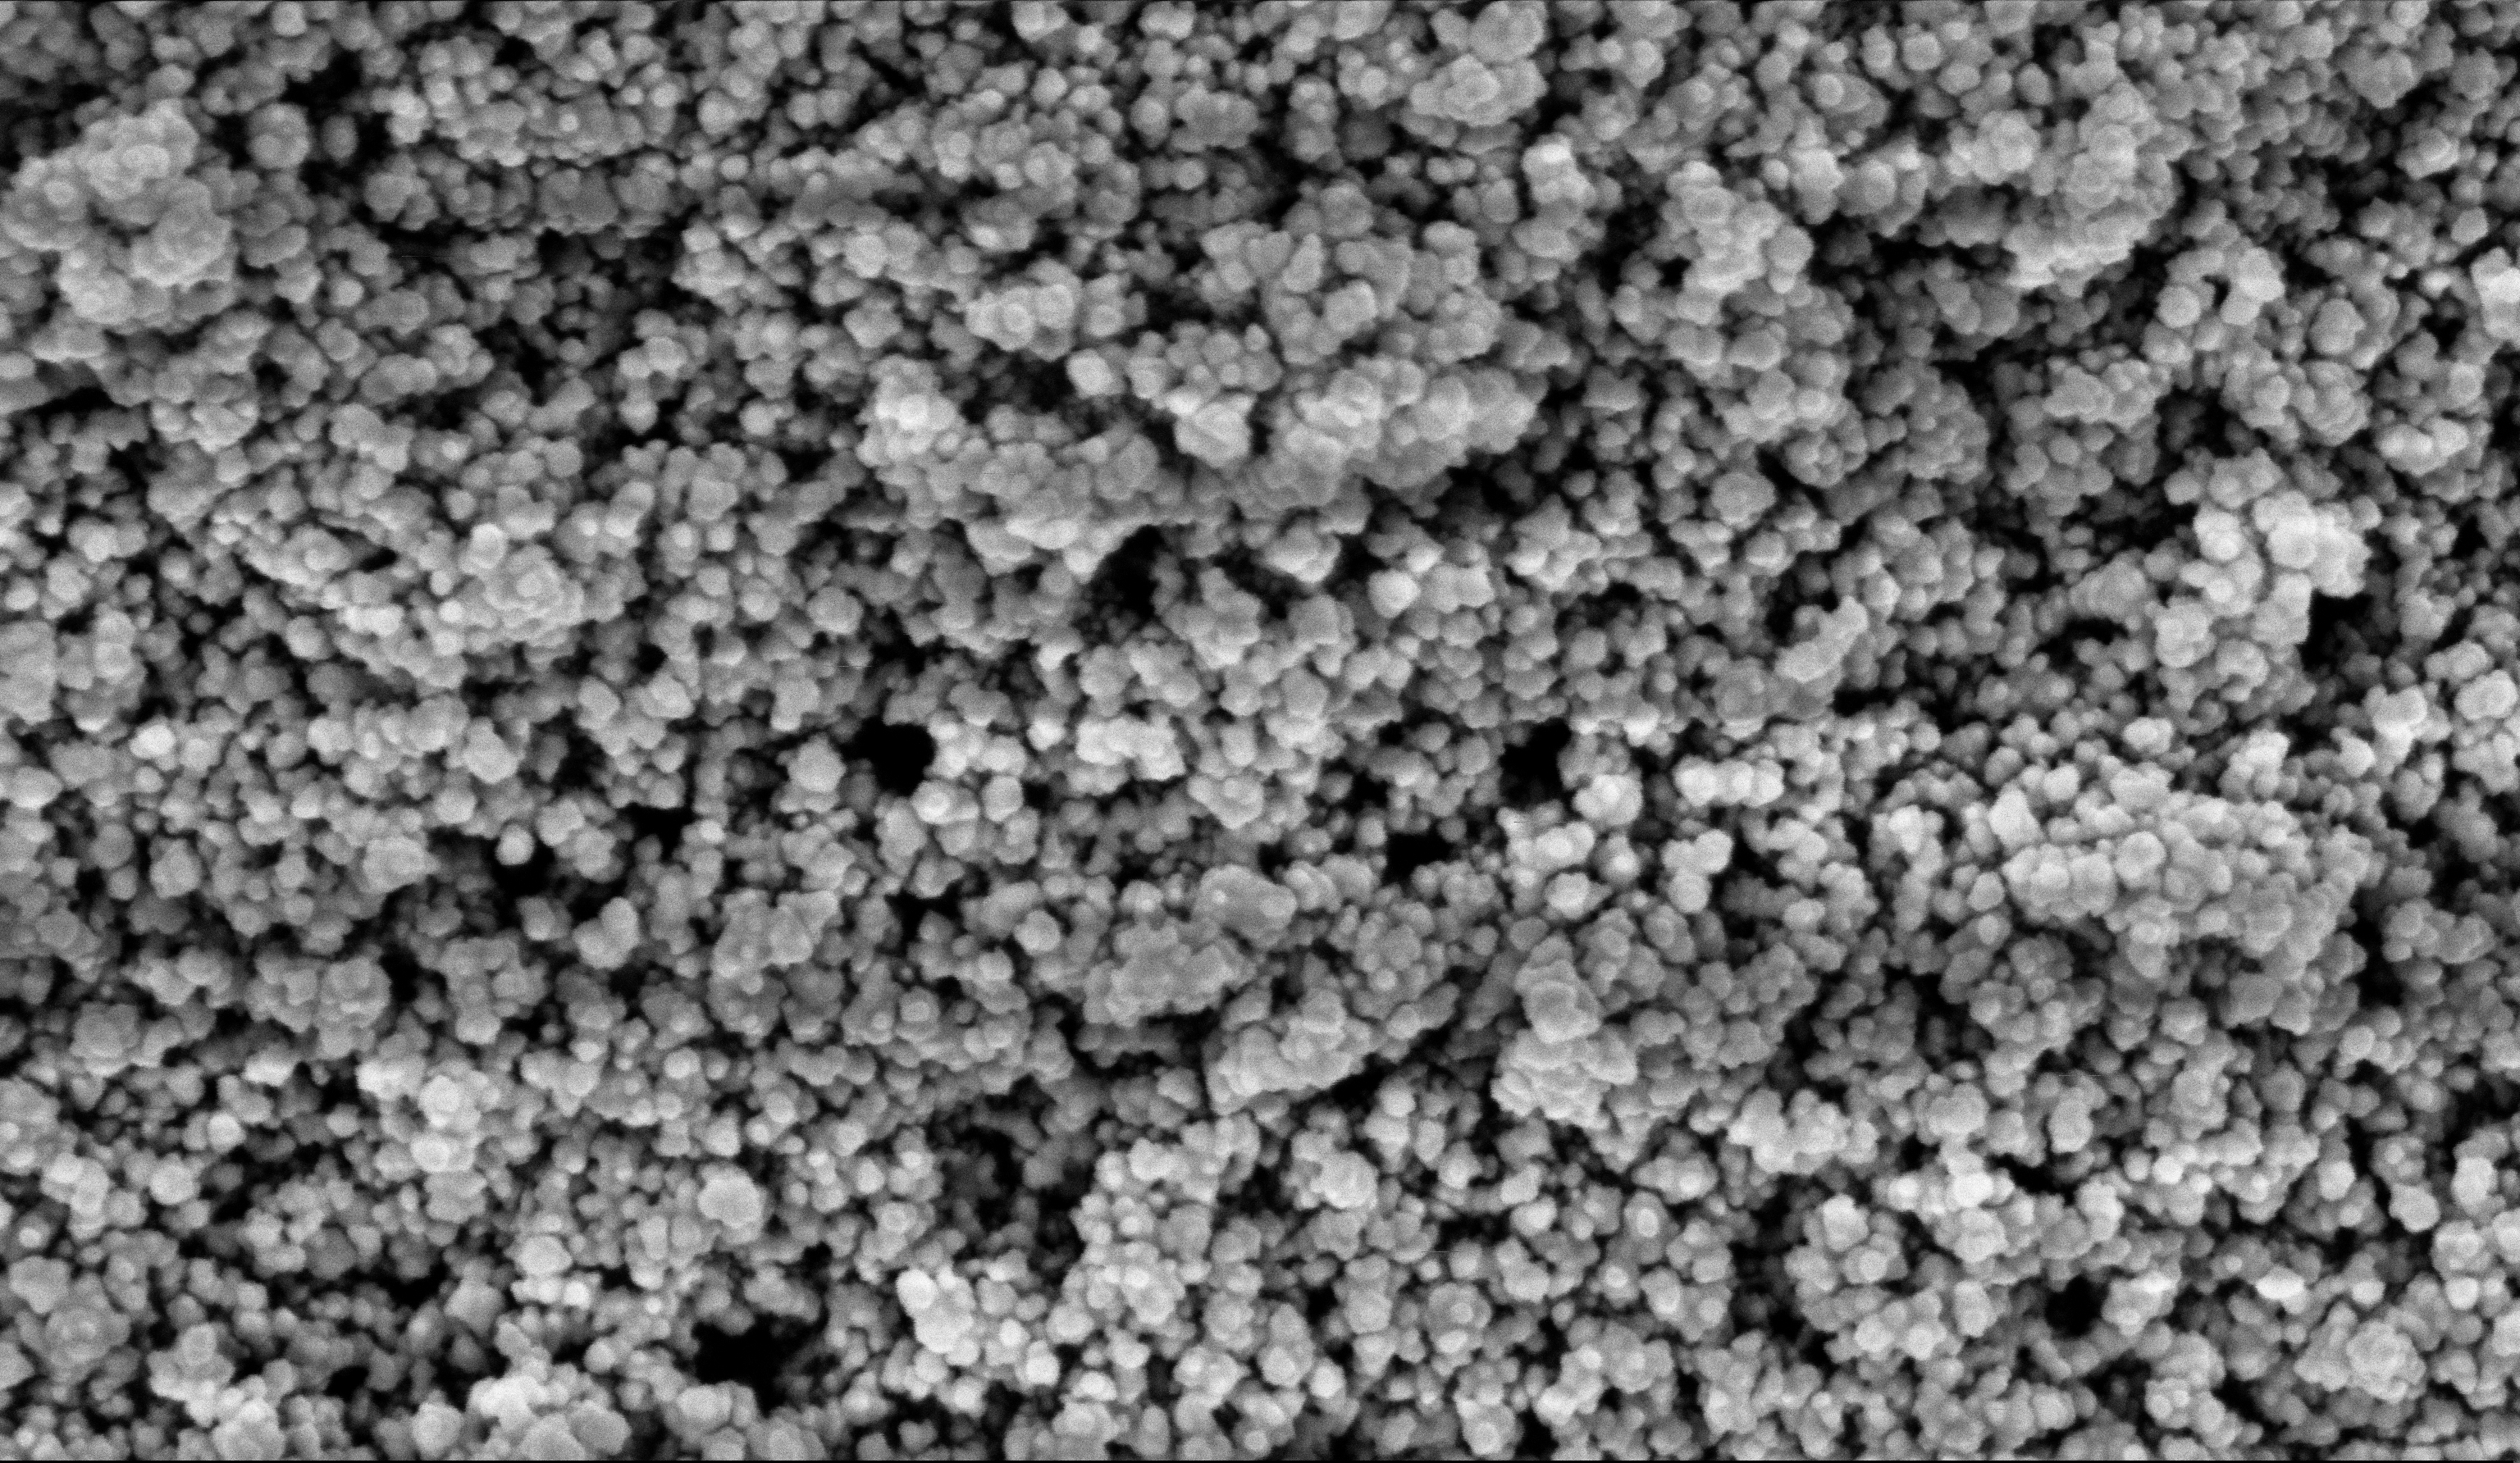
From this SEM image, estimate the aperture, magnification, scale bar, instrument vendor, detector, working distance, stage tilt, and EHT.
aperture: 30 µm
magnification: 135 K X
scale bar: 200 nm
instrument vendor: Zeiss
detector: InLens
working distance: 5.9 mm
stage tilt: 0°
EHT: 5 kV